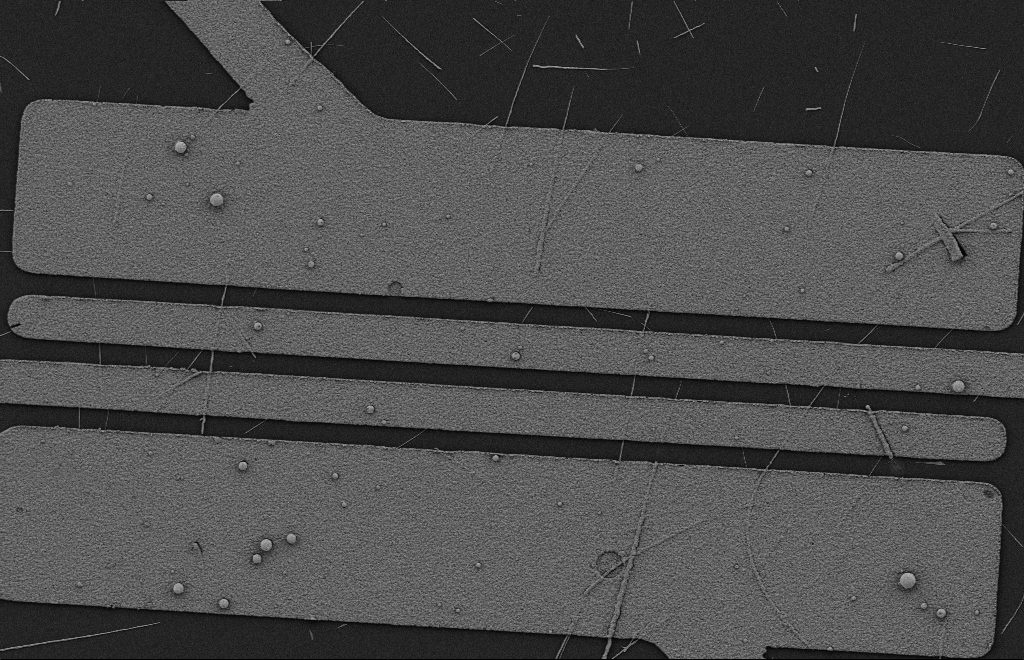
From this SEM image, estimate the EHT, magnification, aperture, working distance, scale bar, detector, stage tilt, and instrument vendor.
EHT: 2 kV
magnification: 6 K X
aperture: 20 µm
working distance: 10 mm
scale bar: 2000 nm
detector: SE2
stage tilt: -0.3°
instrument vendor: Zeiss